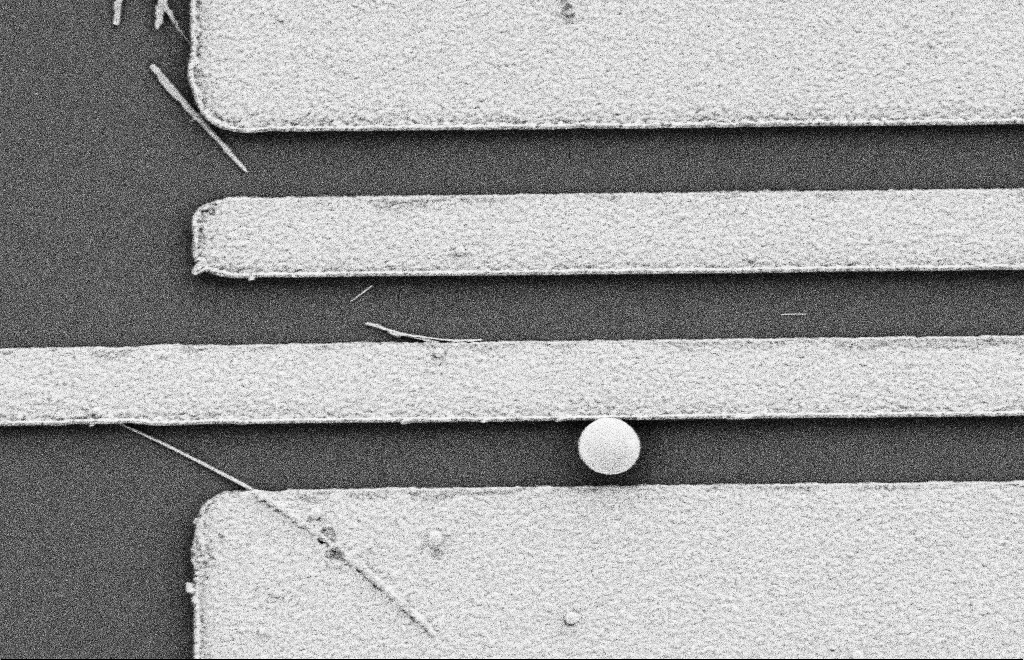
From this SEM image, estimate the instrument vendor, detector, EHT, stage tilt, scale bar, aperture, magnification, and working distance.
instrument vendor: Zeiss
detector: SE2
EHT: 2 kV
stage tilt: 0°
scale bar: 2000 nm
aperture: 20 µm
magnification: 13.57 K X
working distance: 10 mm